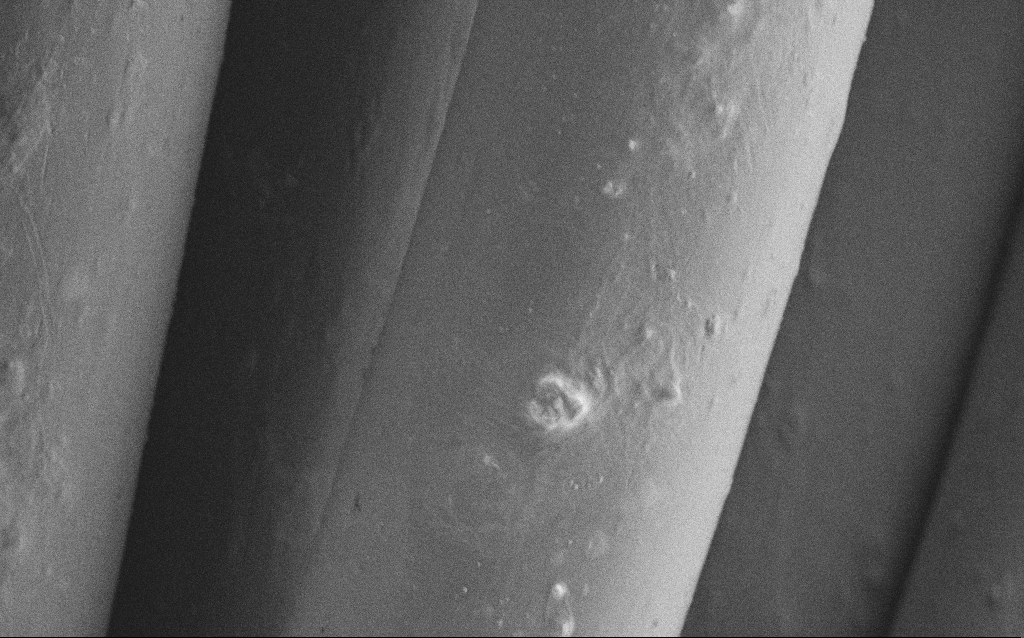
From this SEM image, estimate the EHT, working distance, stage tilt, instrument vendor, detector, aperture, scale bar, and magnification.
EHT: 1 kV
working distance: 4 mm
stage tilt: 0°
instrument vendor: Zeiss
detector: SE2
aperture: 30 µm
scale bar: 10000 nm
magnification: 5.98 K X